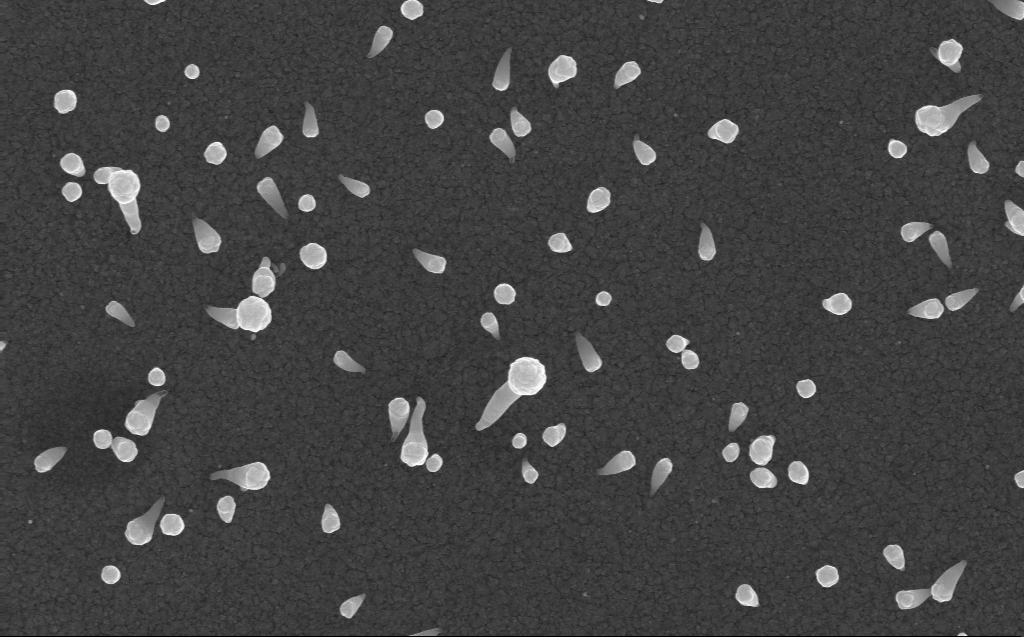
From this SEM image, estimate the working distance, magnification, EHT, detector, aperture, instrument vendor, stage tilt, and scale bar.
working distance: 3 mm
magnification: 54 K X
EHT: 10 kV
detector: InLens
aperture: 30 µm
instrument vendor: Zeiss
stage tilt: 0°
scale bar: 1000 nm